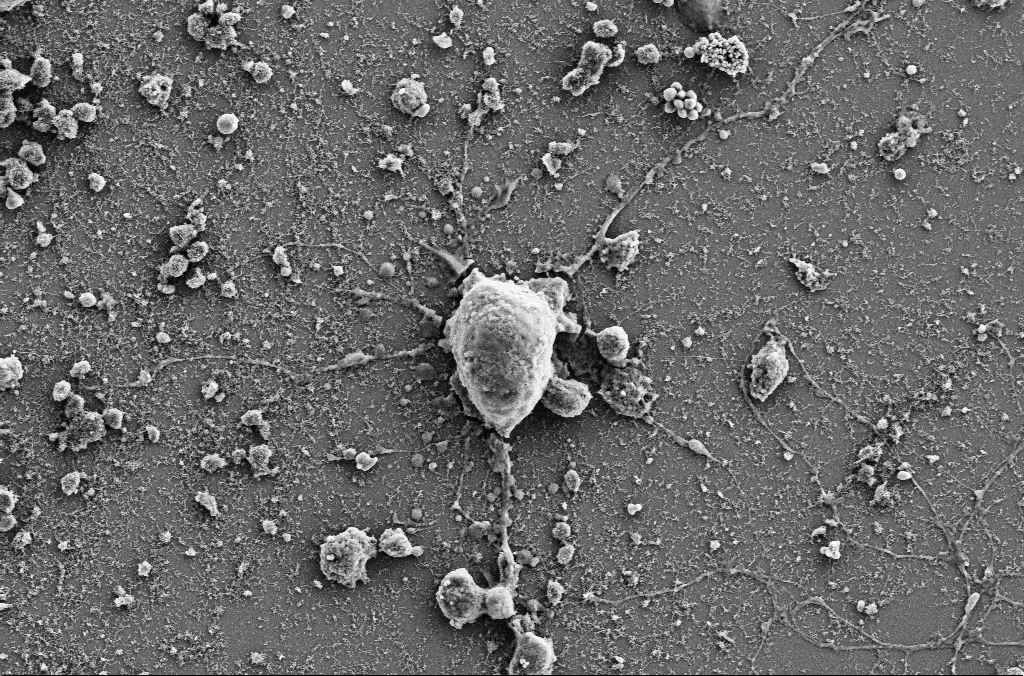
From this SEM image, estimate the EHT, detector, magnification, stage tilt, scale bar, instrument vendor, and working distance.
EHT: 5 kV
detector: SE2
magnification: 4 K X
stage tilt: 0°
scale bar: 10000 nm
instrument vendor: Zeiss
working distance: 4 mm